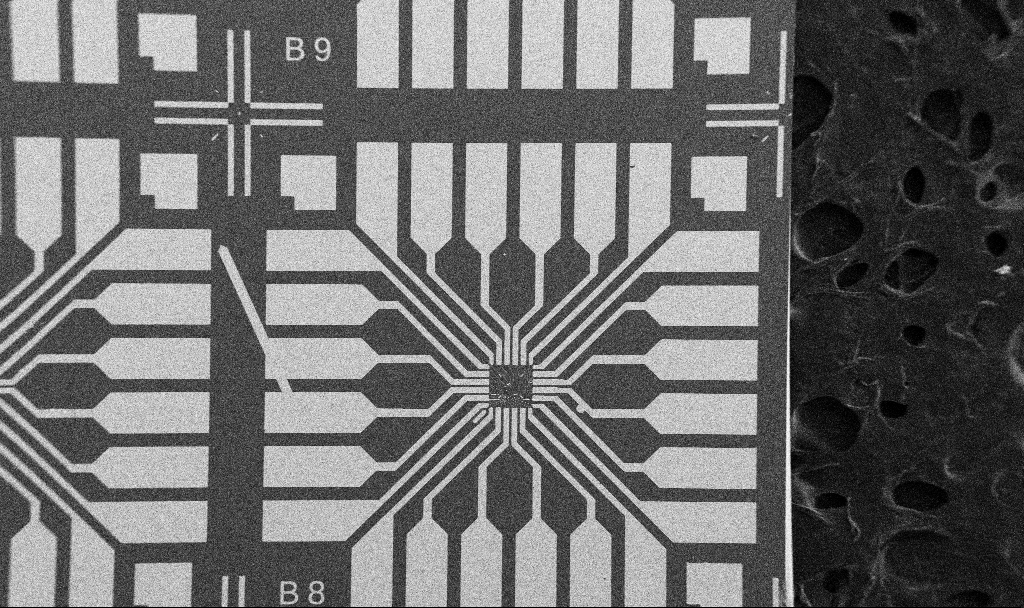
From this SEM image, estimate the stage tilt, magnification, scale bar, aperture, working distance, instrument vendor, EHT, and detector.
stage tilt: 0°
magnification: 0.1 K X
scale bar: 200000 nm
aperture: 30 µm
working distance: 10.7 mm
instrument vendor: Zeiss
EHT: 5 kV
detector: SE2